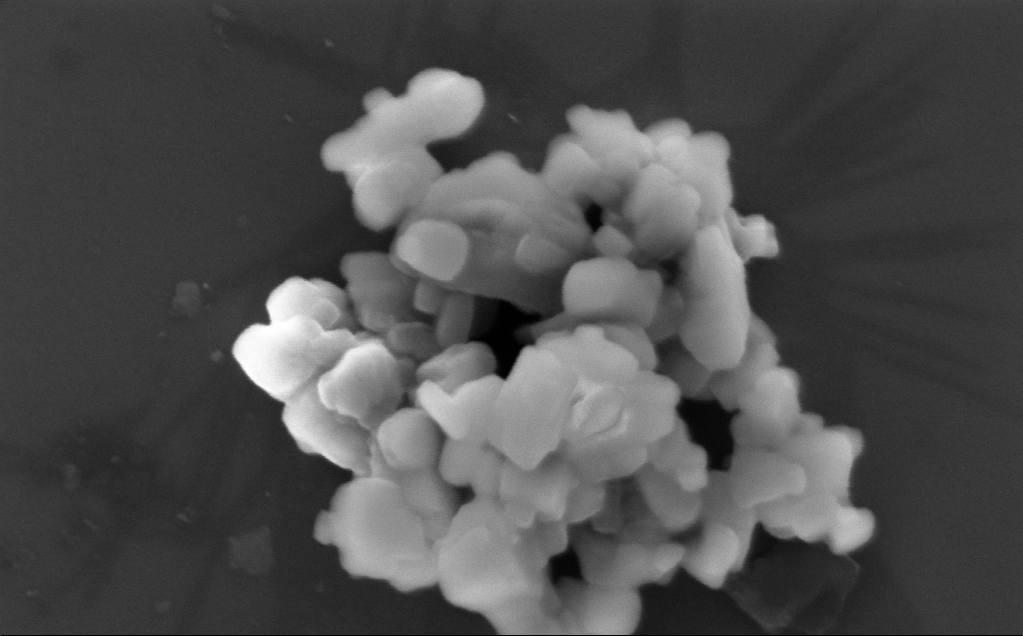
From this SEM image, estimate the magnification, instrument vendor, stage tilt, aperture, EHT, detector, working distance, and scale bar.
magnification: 189.31 K X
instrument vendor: Zeiss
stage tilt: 0°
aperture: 30 µm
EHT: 3 kV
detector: InLens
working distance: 3 mm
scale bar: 200 nm